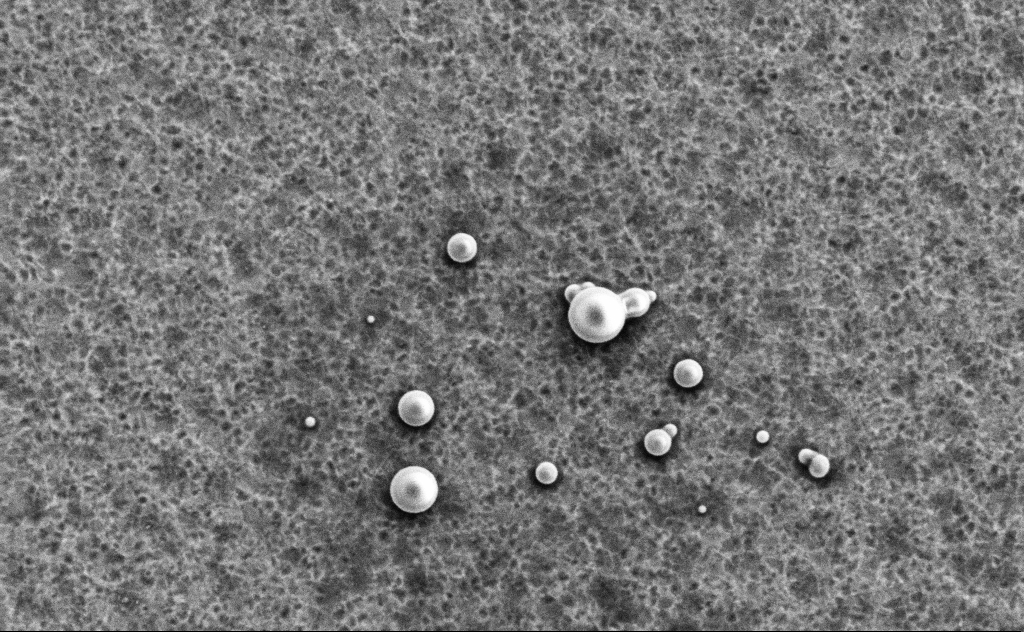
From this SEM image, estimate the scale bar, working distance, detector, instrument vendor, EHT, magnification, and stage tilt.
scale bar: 2000 nm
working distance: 13 mm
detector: SE2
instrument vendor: Zeiss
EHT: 3 kV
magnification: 14.19 K X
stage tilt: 0°